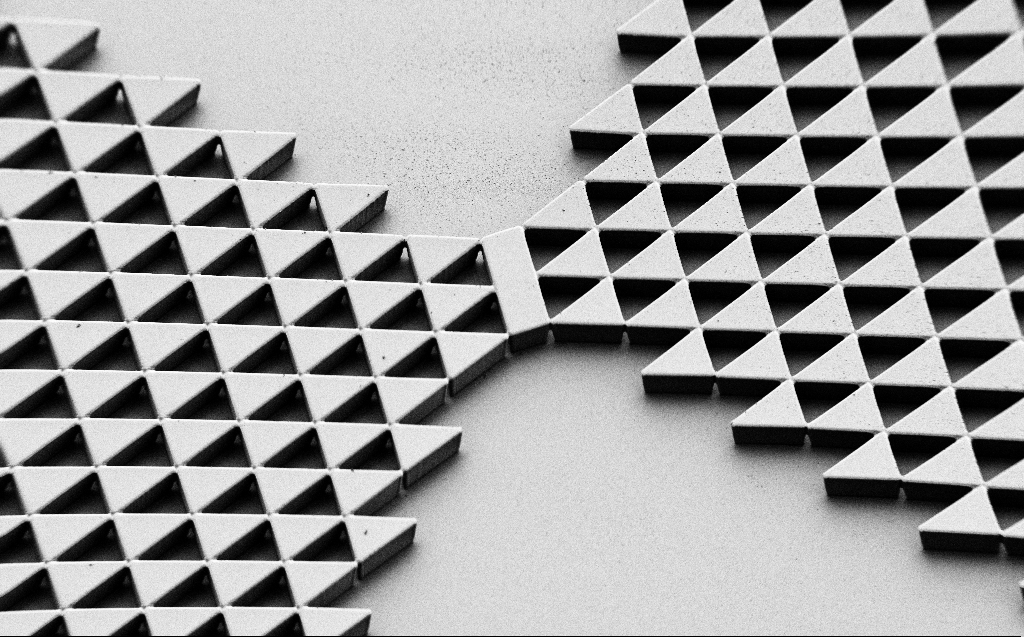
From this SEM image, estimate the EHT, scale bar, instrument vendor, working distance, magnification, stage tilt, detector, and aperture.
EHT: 1 kV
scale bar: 100000 nm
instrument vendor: Zeiss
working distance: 6 mm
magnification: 0.309 K X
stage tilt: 45°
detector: SE2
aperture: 30 µm